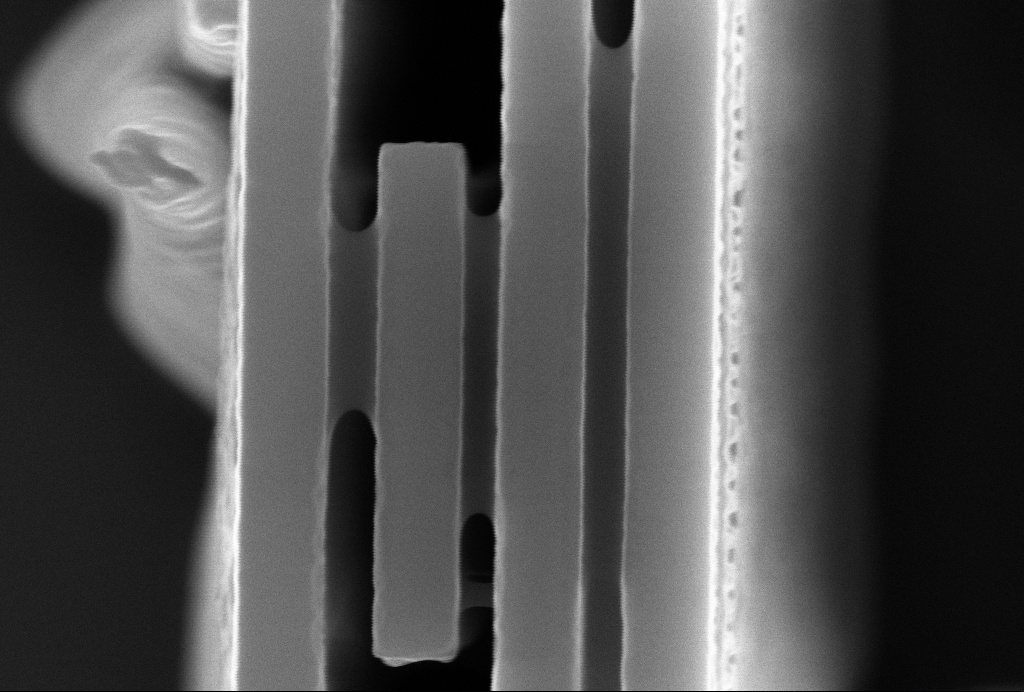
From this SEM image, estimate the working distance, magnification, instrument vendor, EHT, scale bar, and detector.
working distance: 5 mm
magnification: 63.28 K X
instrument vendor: Zeiss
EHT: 10 kV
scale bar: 300 nm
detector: InLens